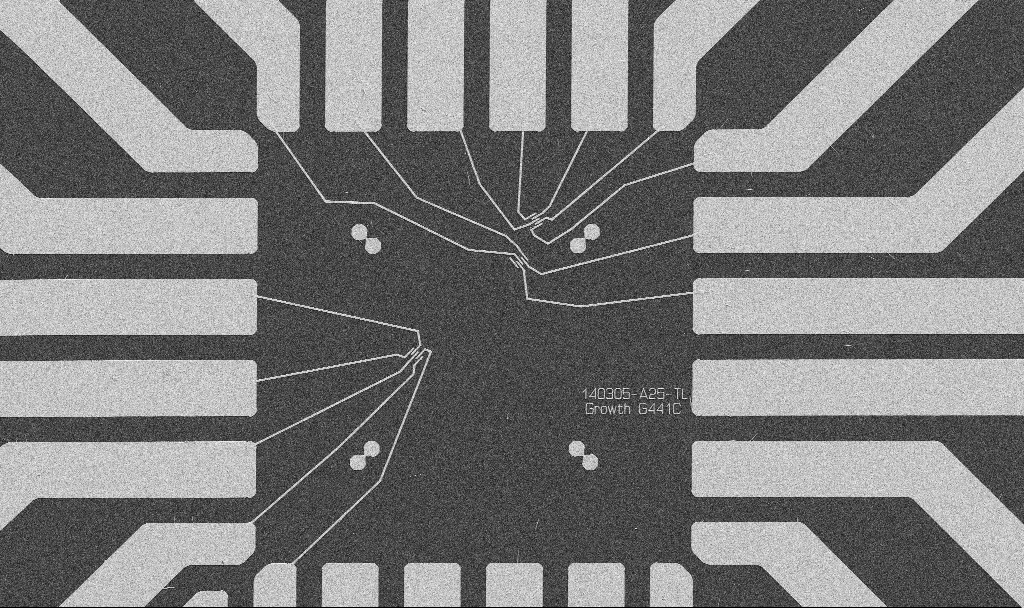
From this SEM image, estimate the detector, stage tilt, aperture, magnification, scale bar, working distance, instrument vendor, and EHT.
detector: SE2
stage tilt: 0°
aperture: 30 µm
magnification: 1 K X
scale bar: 20000 nm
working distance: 10.7 mm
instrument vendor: Zeiss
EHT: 5 kV